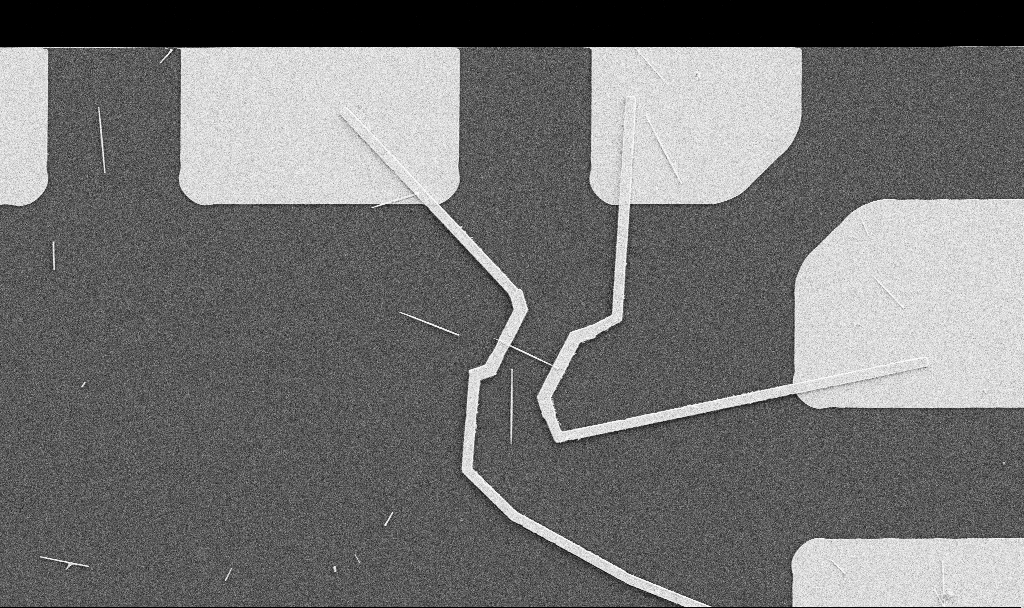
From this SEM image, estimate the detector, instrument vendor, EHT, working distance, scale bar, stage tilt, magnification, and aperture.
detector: SE2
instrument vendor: Zeiss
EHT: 5 kV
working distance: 10.7 mm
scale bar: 10000 nm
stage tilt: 0°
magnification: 5 K X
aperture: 30 µm